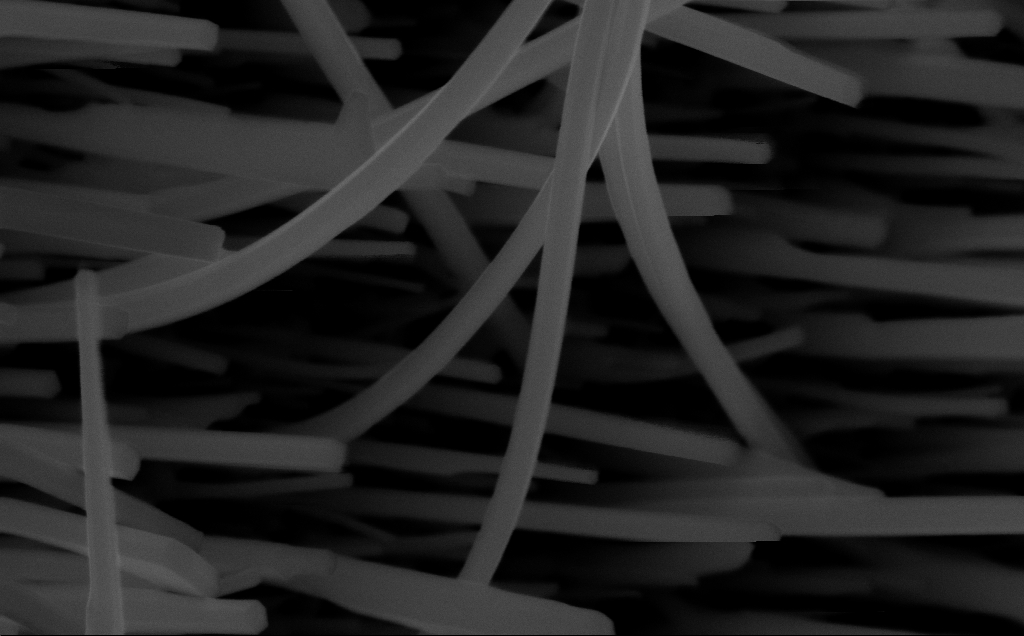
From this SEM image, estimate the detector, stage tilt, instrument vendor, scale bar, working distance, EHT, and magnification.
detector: InLens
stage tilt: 0°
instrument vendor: Zeiss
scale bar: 200 nm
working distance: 6 mm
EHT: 10 kV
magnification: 102.78 K X